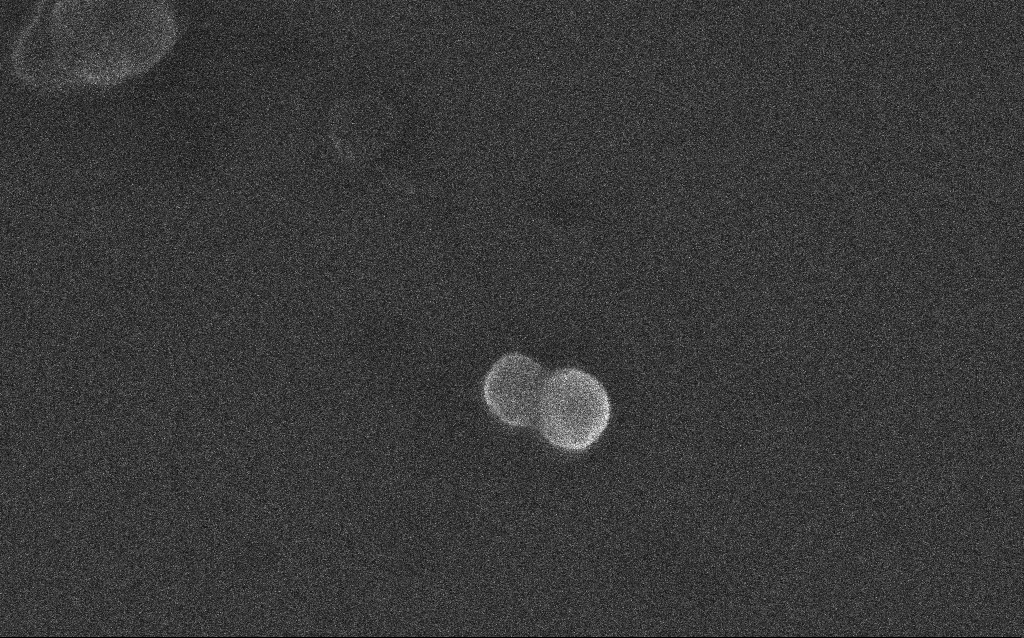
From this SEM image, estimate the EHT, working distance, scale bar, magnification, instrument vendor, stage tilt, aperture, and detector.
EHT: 10 kV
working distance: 3 mm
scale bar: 200 nm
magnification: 196.52 K X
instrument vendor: Zeiss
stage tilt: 0°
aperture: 30 µm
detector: InLens